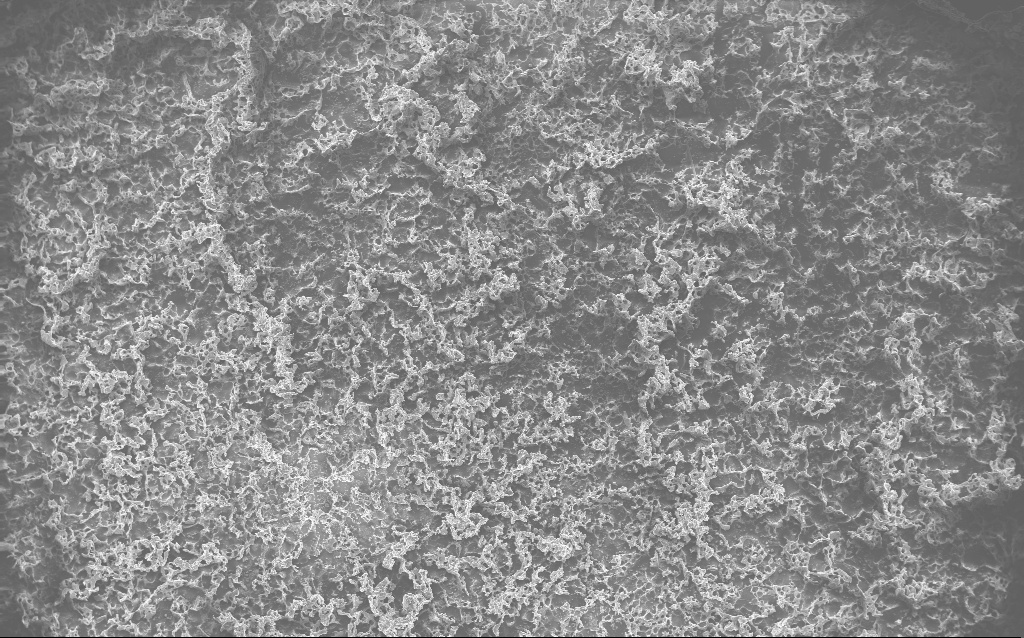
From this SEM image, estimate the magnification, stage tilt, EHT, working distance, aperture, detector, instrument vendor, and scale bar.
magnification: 0.122 K X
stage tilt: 0°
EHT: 10 kV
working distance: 2.7 mm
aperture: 30 µm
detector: InLens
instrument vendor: Zeiss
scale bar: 100000 nm